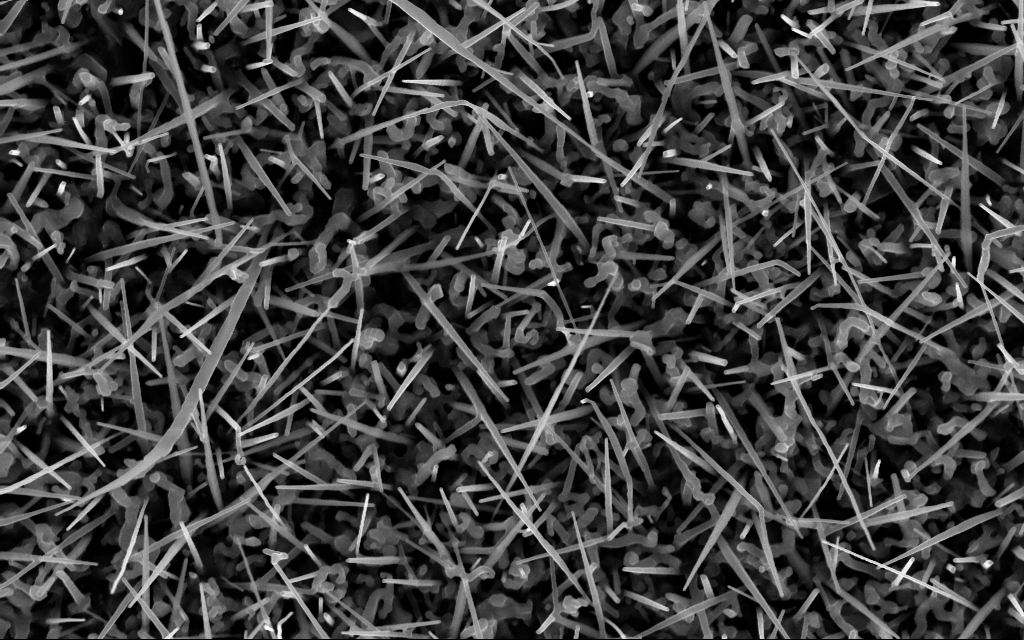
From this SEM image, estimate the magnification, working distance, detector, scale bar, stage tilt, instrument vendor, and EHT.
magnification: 40 K X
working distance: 5 mm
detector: InLens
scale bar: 1000 nm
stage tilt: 0°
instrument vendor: Zeiss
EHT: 10 kV